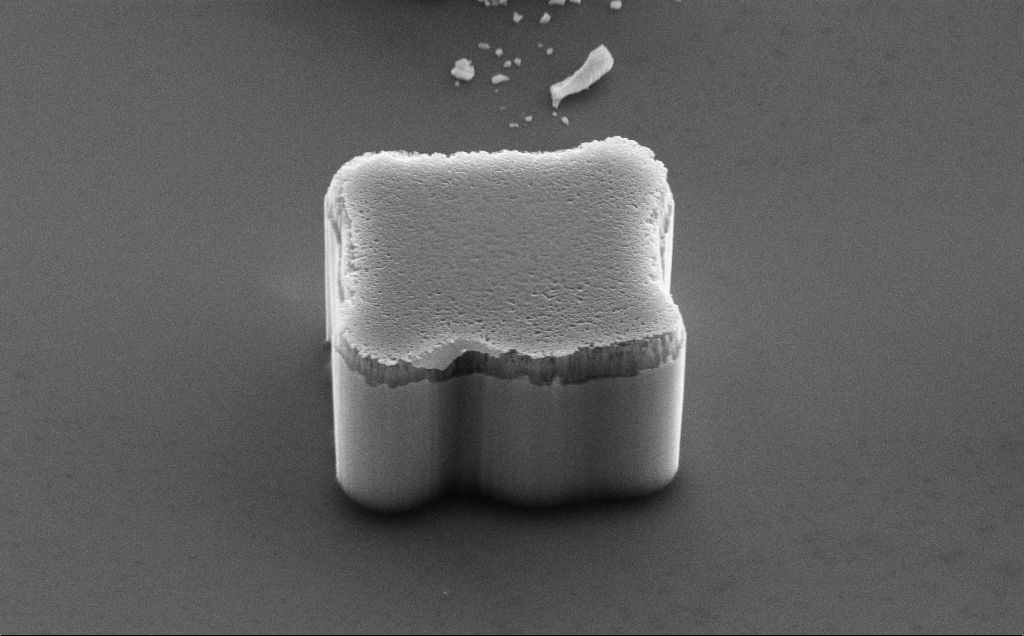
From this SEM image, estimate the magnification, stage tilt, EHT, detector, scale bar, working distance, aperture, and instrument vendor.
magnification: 15.56 K X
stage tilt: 50.6°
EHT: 10 kV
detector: SE2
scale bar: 2000 nm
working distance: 13 mm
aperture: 30 µm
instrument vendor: Zeiss